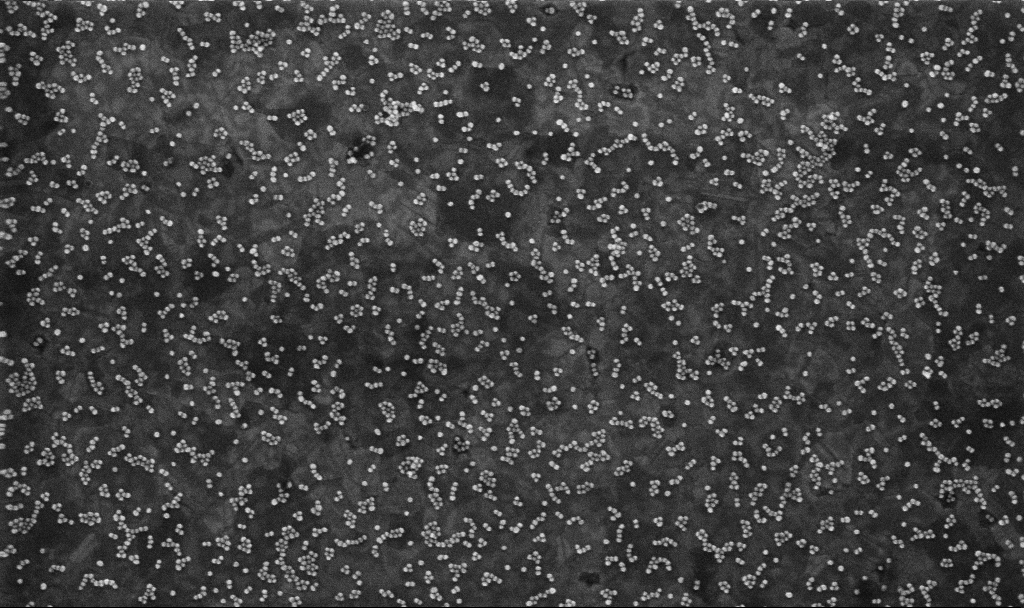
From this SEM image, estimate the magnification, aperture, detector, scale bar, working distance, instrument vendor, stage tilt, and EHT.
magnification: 100 K X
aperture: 30 µm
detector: InLens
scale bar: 200 nm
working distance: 3.8 mm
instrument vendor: Zeiss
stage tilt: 0°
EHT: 10 kV